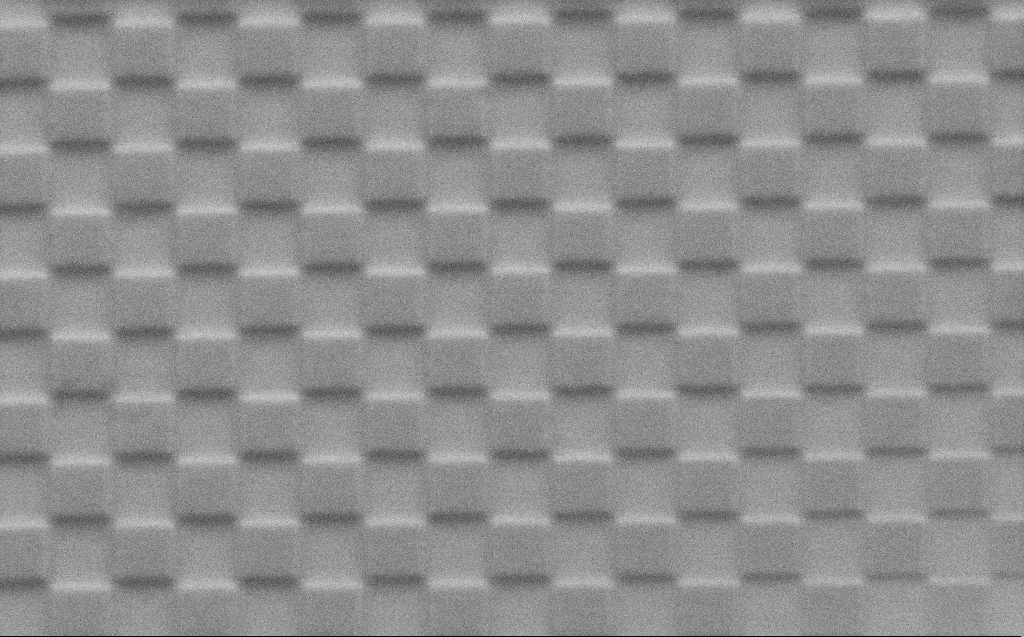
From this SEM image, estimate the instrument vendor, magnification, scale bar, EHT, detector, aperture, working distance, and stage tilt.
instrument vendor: Zeiss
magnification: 23.44 K X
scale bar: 2000 nm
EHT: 2 kV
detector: SE2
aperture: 30 µm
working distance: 4 mm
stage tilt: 45°